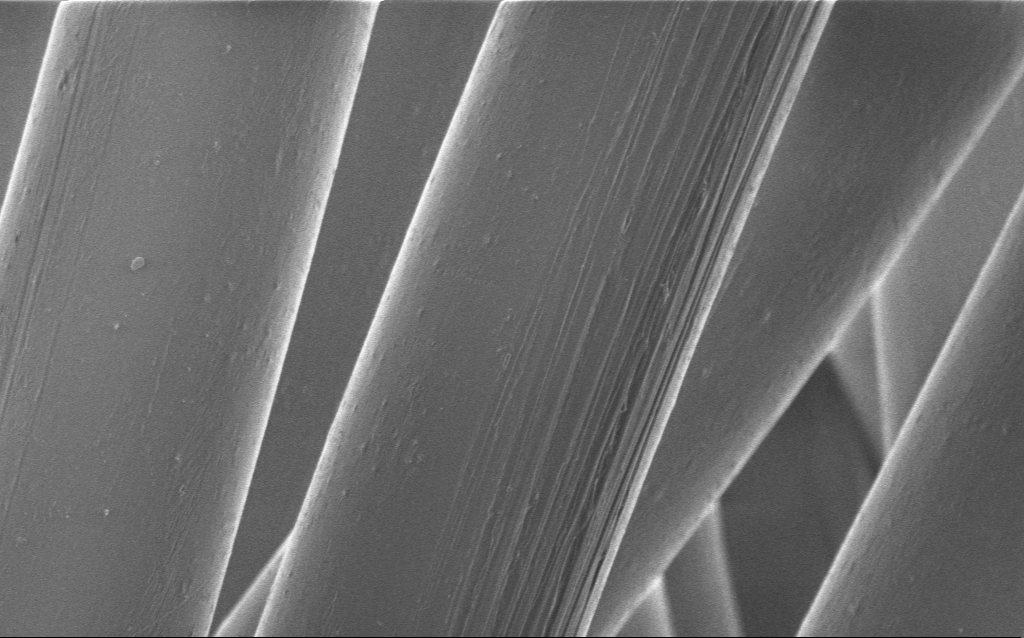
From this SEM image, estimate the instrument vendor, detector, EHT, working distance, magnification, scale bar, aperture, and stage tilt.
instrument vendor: Zeiss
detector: InLens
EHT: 1 kV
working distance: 4 mm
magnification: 4.81 K X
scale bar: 10000 nm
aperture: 30 µm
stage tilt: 0°